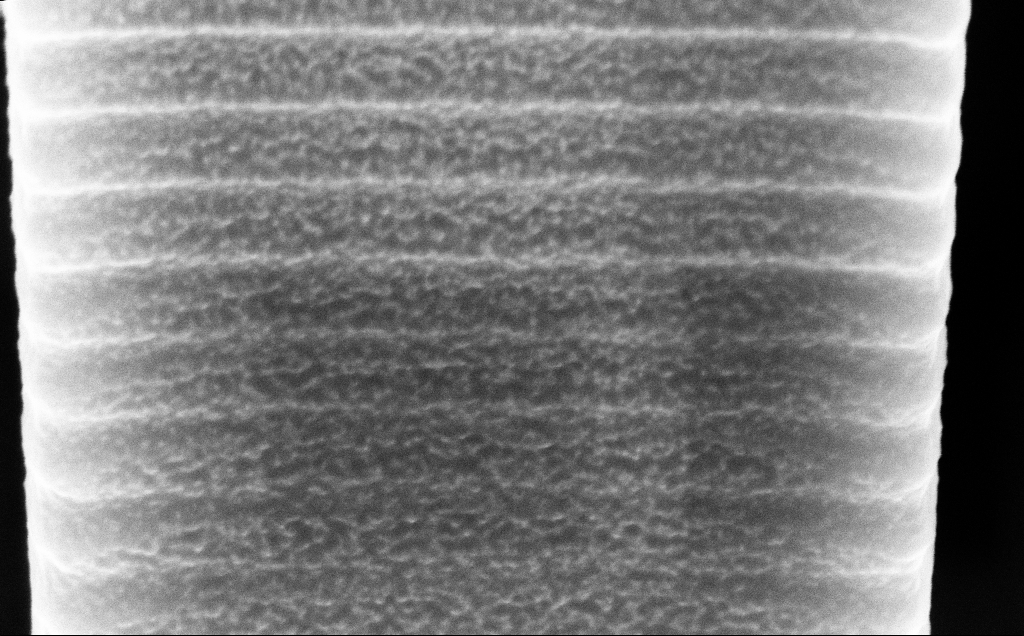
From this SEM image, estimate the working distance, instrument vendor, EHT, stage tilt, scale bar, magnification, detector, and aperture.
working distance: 5 mm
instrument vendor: Zeiss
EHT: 10 kV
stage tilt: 45°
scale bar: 200 nm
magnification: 78.02 K X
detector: InLens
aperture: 30 µm